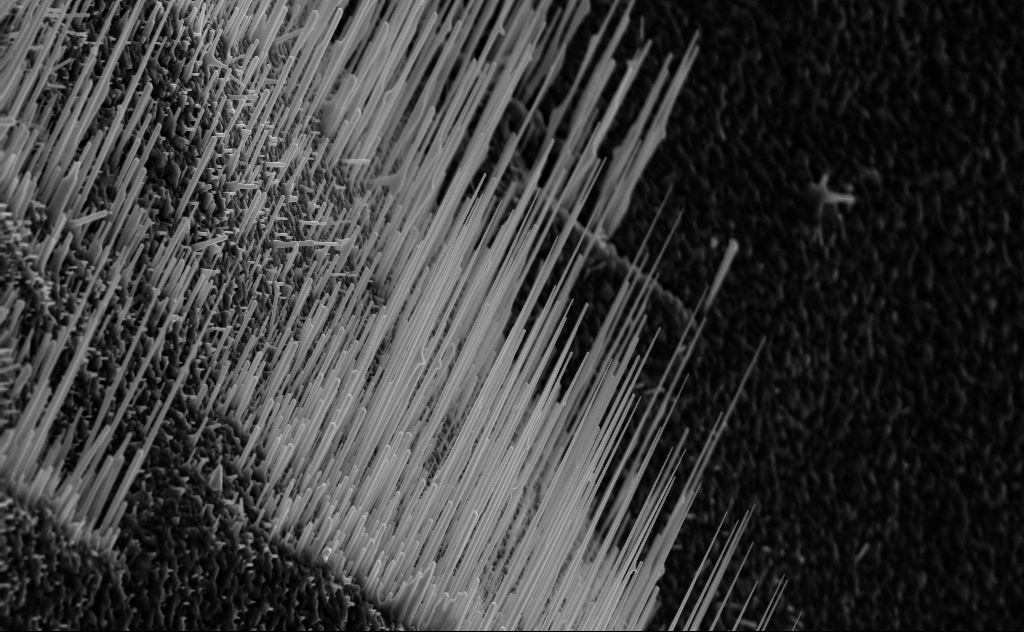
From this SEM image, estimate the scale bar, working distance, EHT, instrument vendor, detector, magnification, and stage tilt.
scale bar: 2000 nm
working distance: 7 mm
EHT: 10 kV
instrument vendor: Zeiss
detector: InLens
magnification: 10.26 K X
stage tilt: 0°